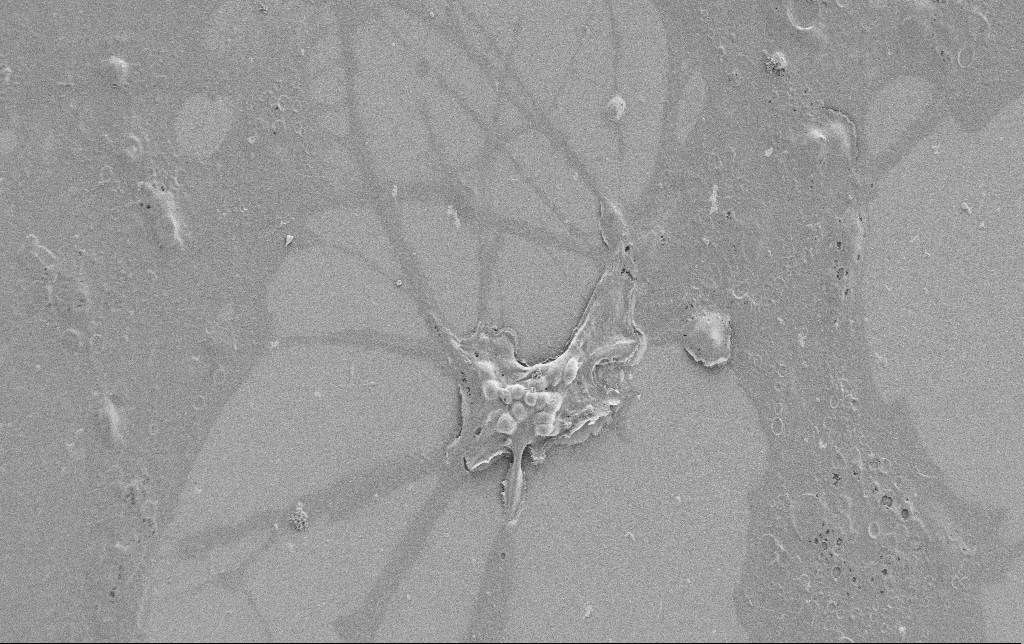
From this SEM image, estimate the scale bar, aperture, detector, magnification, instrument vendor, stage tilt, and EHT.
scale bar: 20000 nm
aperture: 30 µm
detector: SE2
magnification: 1 K X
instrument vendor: Zeiss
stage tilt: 0°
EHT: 1 kV